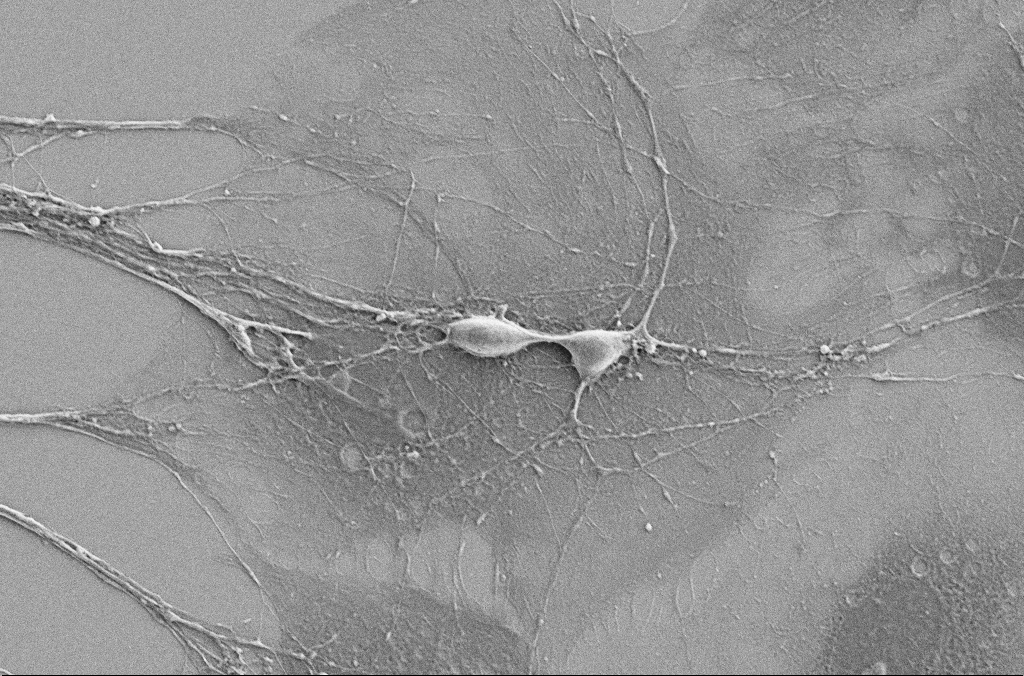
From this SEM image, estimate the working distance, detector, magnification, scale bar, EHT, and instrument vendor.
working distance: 5.9 mm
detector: SE2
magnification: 2.5 K X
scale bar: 10000 nm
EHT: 5 kV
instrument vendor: Zeiss